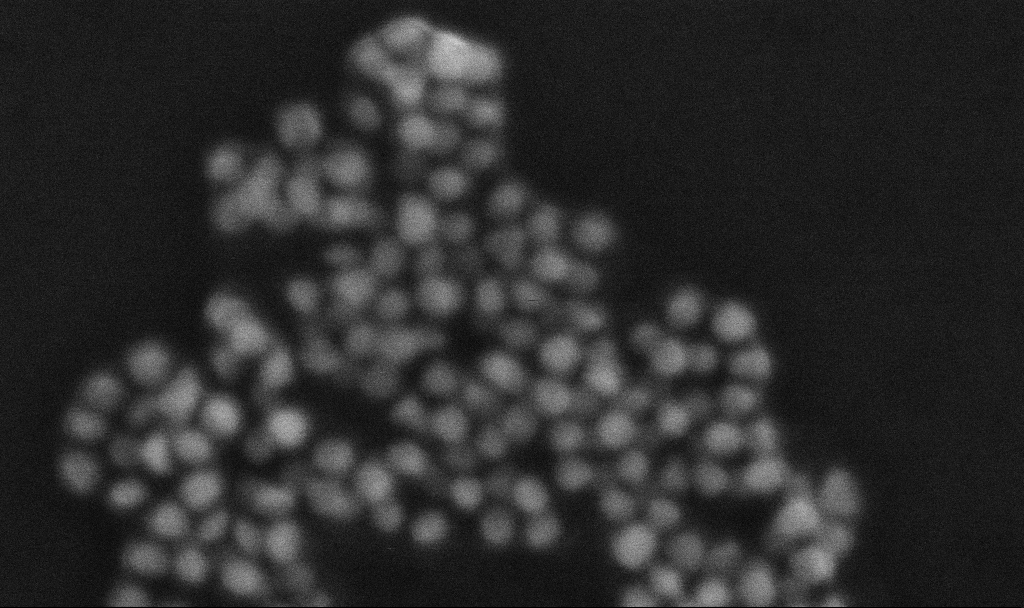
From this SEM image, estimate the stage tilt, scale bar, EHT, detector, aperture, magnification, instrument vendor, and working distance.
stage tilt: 0°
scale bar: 20 nm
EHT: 10 kV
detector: InLens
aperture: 30 µm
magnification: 736.15 K X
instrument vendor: Zeiss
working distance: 3.3 mm